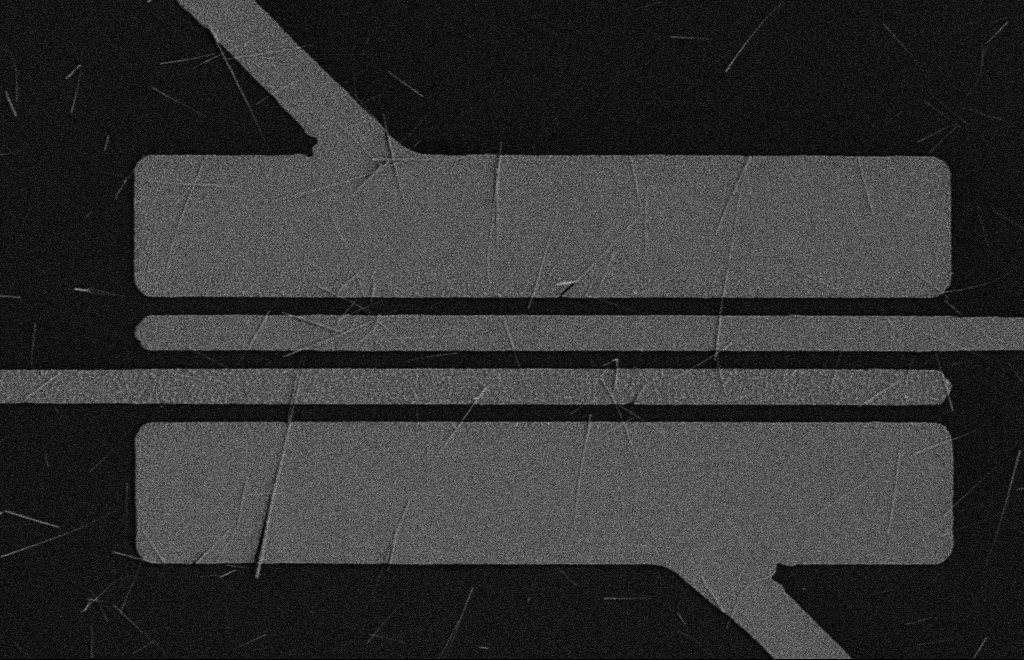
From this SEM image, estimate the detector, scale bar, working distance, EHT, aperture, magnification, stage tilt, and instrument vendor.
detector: SE2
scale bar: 2000 nm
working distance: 16 mm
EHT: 5 kV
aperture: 10 µm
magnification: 4.96 K X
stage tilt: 0°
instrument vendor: Zeiss